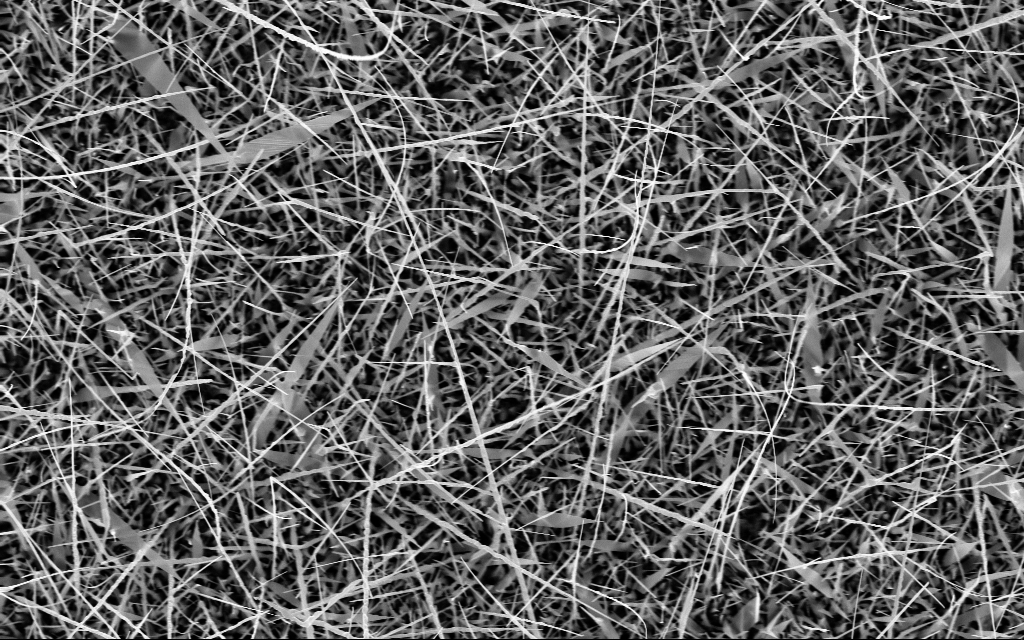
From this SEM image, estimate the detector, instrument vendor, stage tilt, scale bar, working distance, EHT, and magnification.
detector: InLens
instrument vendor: Zeiss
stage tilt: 0°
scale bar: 2000 nm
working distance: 6 mm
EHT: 10 kV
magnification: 10 K X